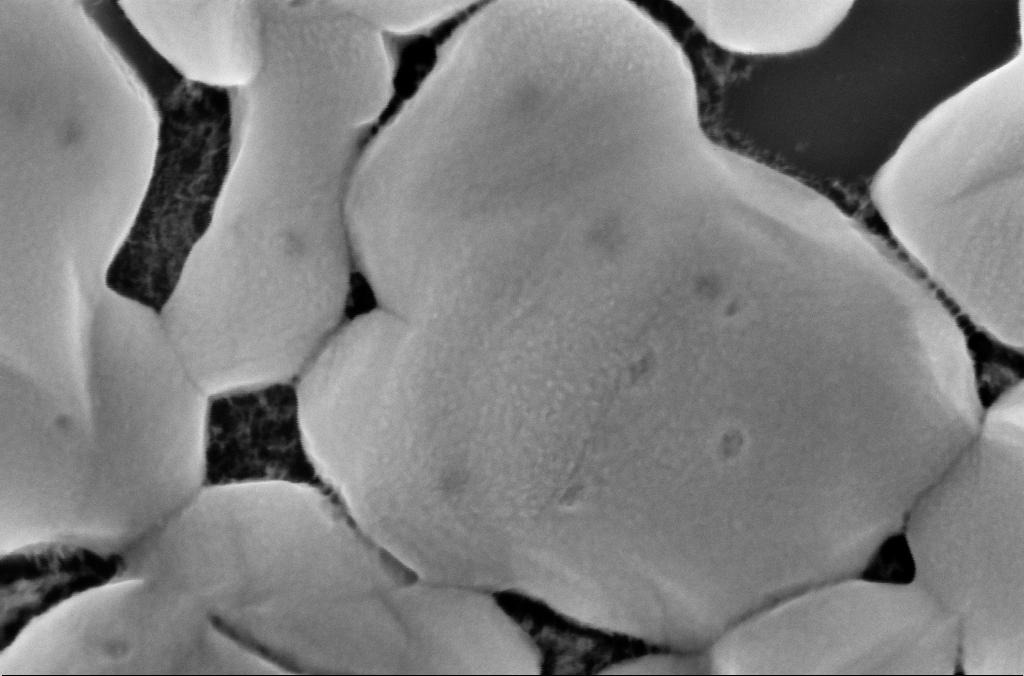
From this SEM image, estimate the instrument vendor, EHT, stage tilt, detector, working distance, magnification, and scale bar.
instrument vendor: Zeiss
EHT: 5 kV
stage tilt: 0°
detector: InLens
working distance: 3 mm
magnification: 250 K X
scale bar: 100 nm